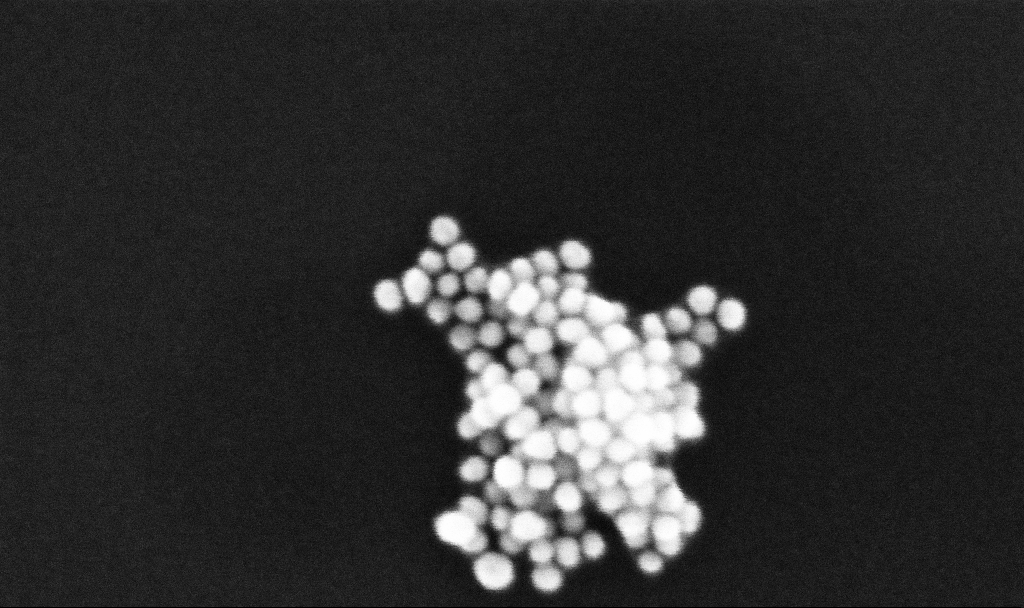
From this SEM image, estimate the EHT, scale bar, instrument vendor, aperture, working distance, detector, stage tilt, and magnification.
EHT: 10 kV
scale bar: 100 nm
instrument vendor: Zeiss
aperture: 30 µm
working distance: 3.2 mm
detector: InLens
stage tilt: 0°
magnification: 513.18 K X